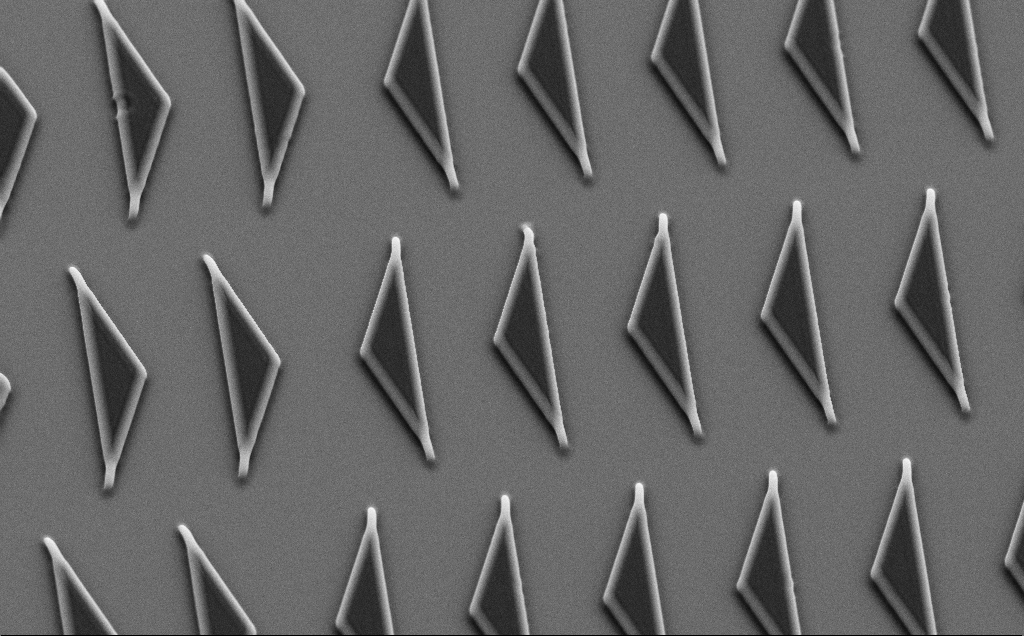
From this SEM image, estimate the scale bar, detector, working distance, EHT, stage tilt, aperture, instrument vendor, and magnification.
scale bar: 20000 nm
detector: SE2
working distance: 8 mm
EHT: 10 kV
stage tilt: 35°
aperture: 30 µm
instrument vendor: Zeiss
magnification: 2.92 K X